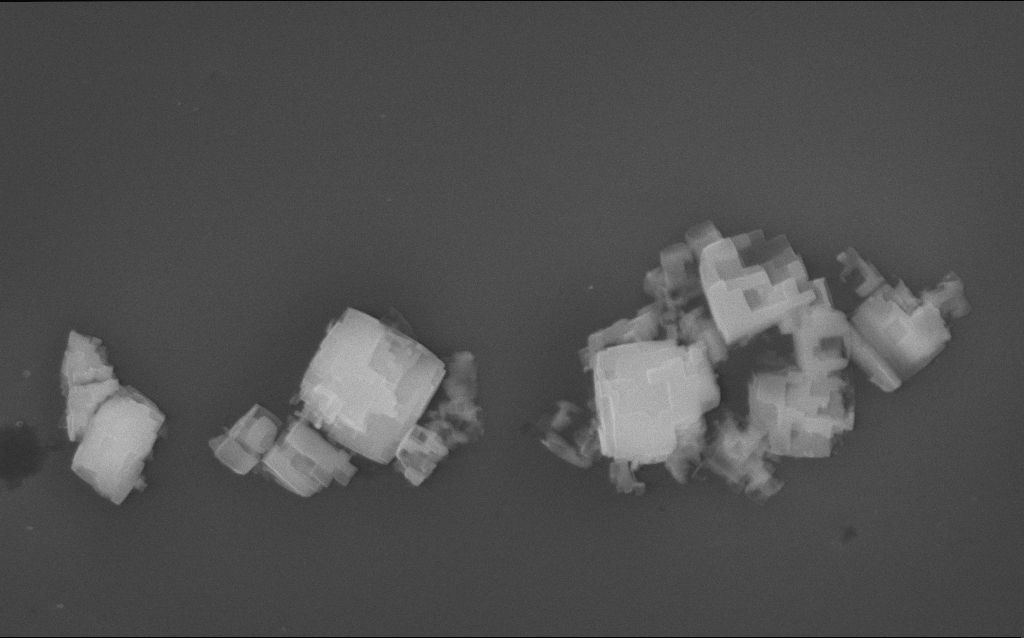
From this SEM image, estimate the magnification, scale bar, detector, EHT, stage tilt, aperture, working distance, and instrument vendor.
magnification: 42.81 K X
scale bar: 1000 nm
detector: InLens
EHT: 10 kV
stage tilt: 0°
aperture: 30 µm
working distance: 2 mm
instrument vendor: Zeiss